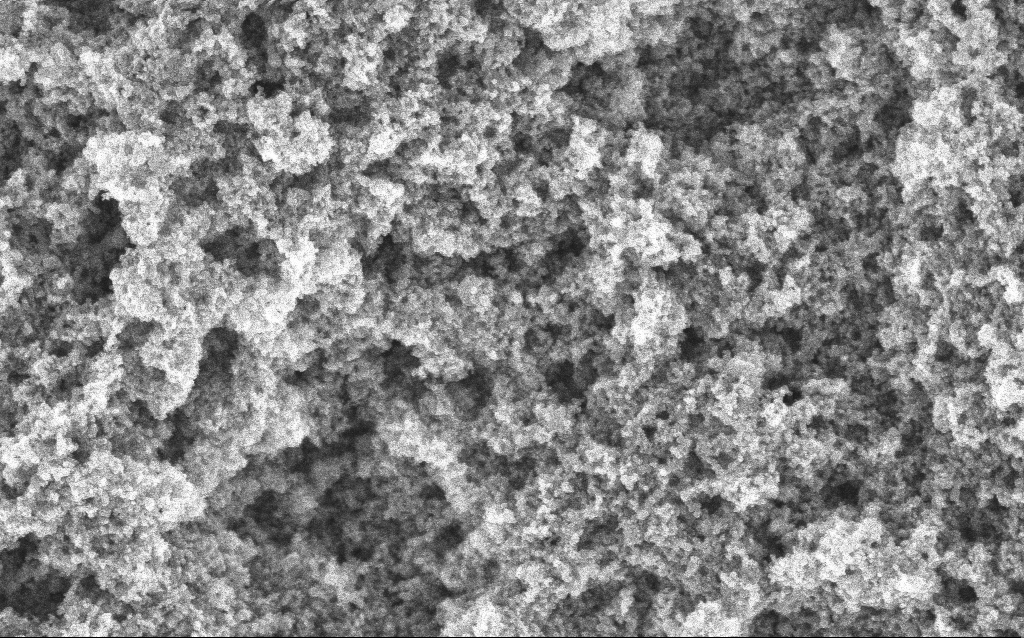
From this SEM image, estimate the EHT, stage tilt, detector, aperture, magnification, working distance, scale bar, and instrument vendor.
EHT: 5 kV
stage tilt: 0°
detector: InLens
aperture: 30 µm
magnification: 65.04 K X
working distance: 4.4 mm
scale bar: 1000 nm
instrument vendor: Zeiss